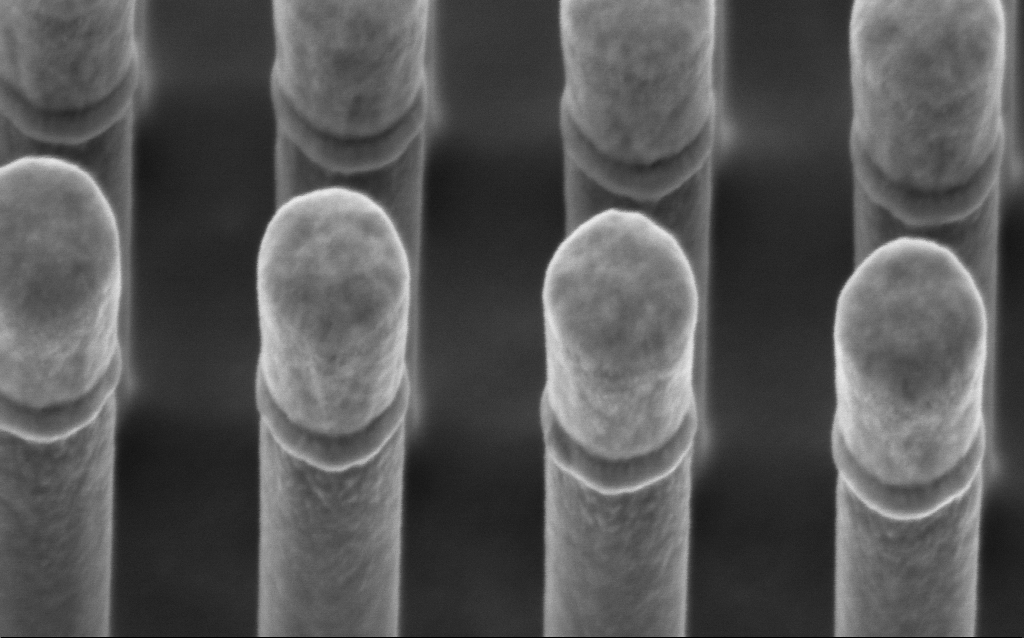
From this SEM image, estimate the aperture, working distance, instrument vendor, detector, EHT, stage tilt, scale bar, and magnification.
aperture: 30 µm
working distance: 2.4 mm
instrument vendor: Zeiss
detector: InLens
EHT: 2 kV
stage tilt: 45°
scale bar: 200 nm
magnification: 213.67 K X